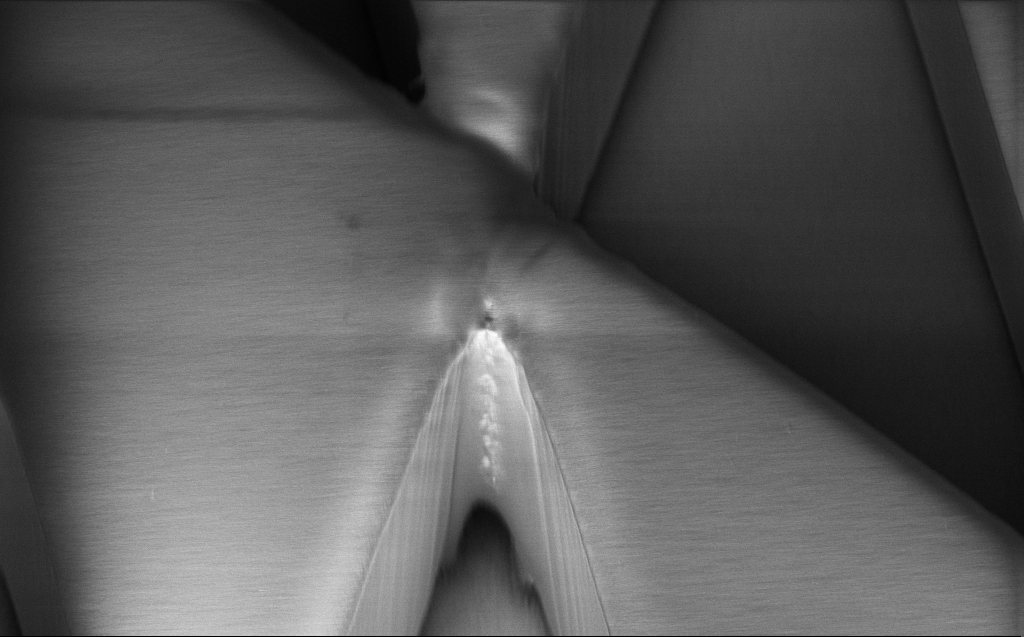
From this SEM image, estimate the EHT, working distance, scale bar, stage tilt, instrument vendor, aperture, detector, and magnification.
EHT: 1 kV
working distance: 6 mm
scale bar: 10000 nm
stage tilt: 45°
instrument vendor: Zeiss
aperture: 30 µm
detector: InLens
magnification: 5.31 K X